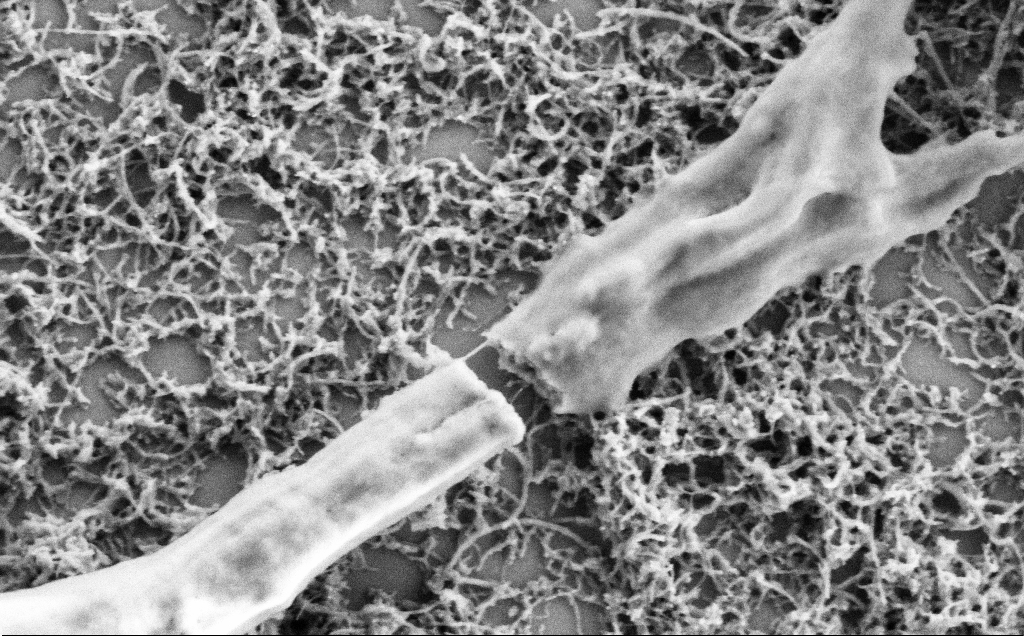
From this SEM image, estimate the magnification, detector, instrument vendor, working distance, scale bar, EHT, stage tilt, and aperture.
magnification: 50 K X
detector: SE2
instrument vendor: Zeiss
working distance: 7.1 mm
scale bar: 1000 nm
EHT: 2 kV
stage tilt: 0°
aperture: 30 µm